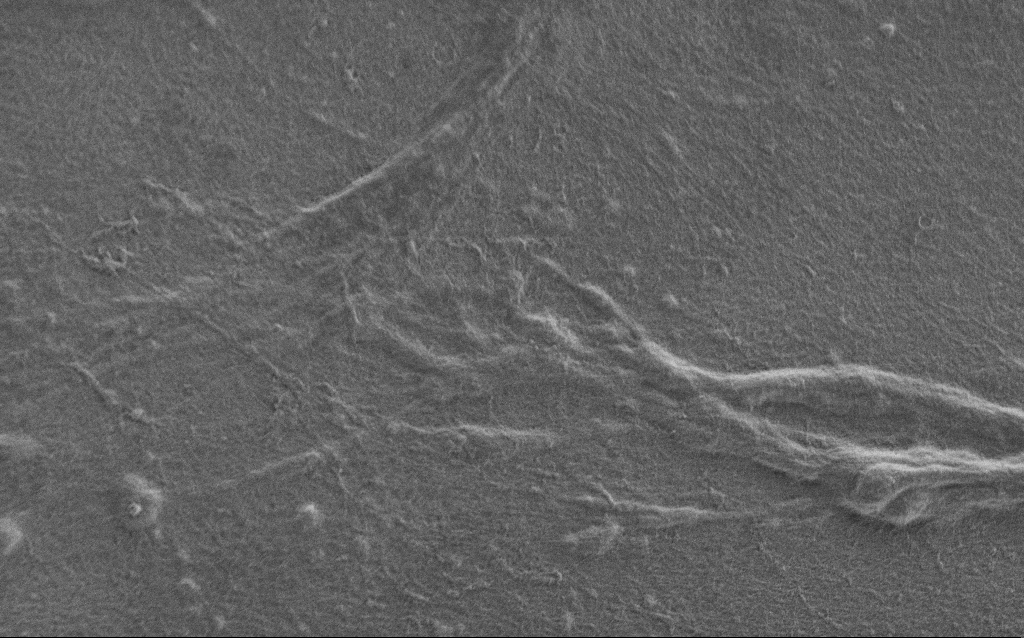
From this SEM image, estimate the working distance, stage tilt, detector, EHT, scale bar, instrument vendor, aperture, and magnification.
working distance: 6 mm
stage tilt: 0°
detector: SE2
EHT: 0.9 kV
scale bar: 2000 nm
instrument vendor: Zeiss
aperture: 30 µm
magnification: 7.5 K X